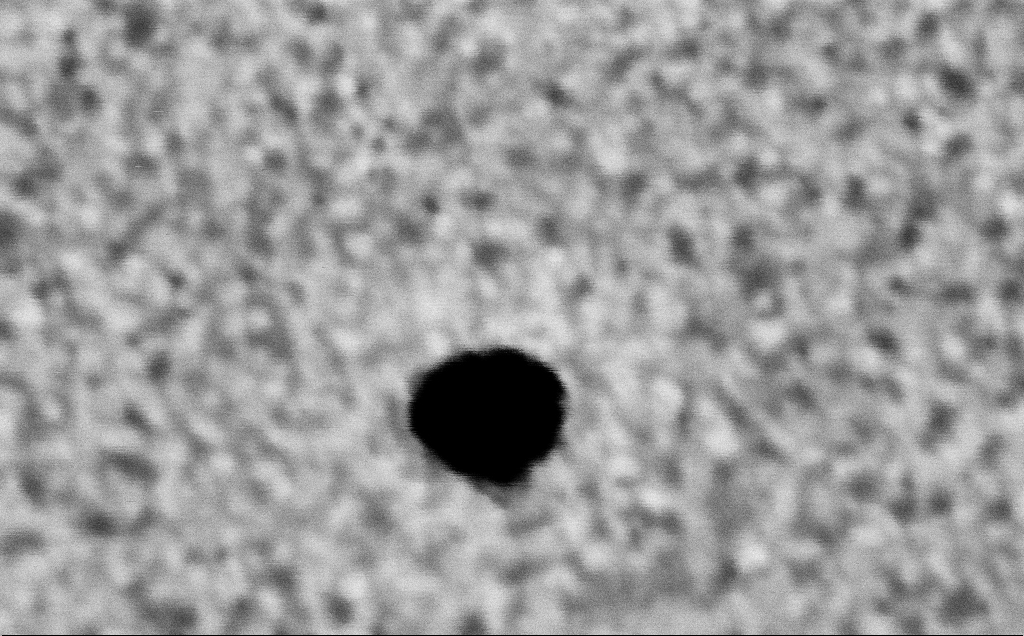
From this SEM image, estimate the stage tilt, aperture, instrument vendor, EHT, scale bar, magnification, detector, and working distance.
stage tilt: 0°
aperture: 30 µm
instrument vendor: Zeiss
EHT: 3 kV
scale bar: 20 nm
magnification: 823.1 K X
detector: InLens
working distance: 3.2 mm